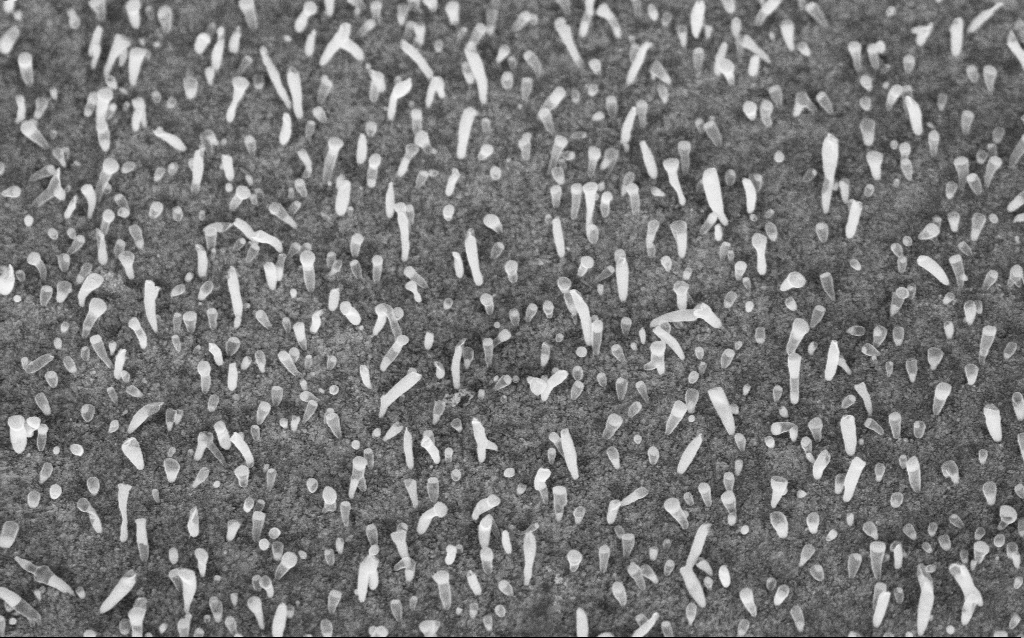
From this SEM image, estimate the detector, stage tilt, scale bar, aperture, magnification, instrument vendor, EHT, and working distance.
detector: InLens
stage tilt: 45°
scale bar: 1000 nm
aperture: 30 µm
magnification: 50 K X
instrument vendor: Zeiss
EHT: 5 kV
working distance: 6.5 mm